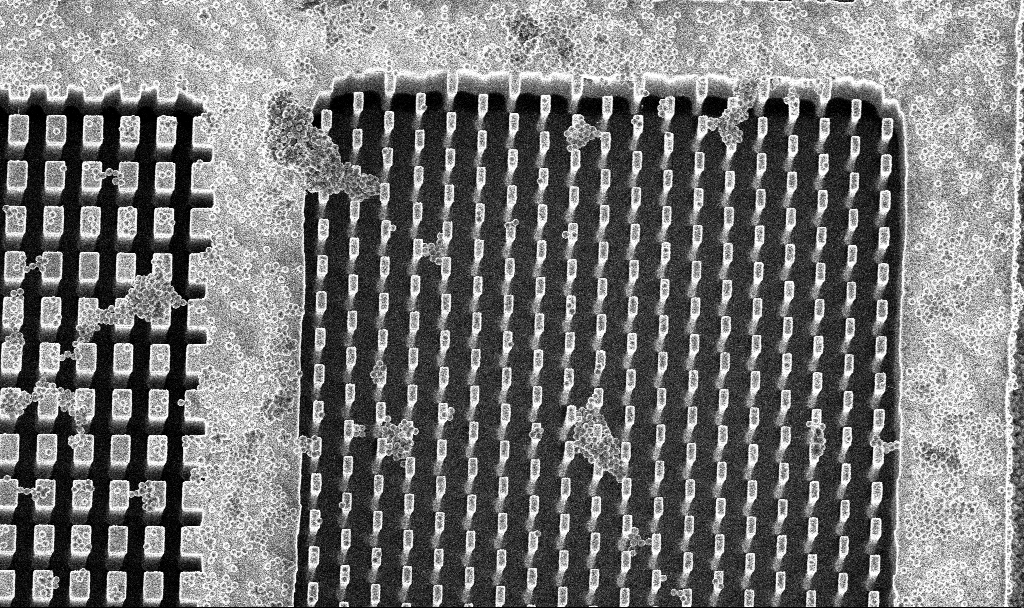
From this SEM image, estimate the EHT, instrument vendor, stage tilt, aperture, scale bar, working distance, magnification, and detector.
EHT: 5 kV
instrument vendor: Zeiss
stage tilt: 8°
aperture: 30 µm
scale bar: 10000 nm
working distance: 3.3 mm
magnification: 4.19 K X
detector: InLens